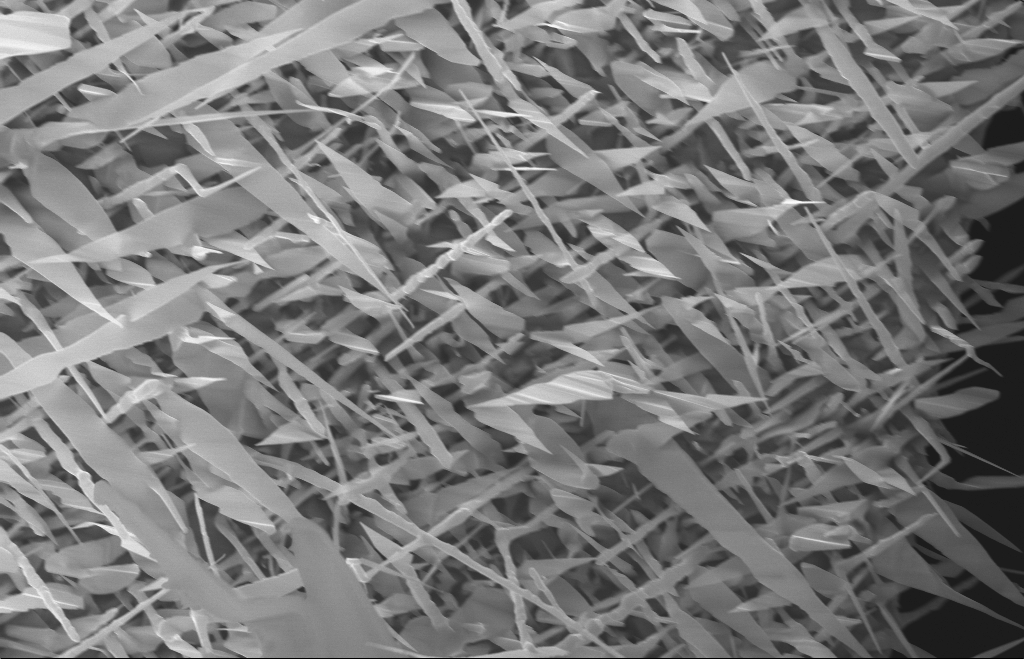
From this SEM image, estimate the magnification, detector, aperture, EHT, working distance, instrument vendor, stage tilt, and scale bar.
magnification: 20 K X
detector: InLens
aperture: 30 µm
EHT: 10 kV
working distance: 8 mm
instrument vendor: Zeiss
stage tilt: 0°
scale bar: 1000 nm